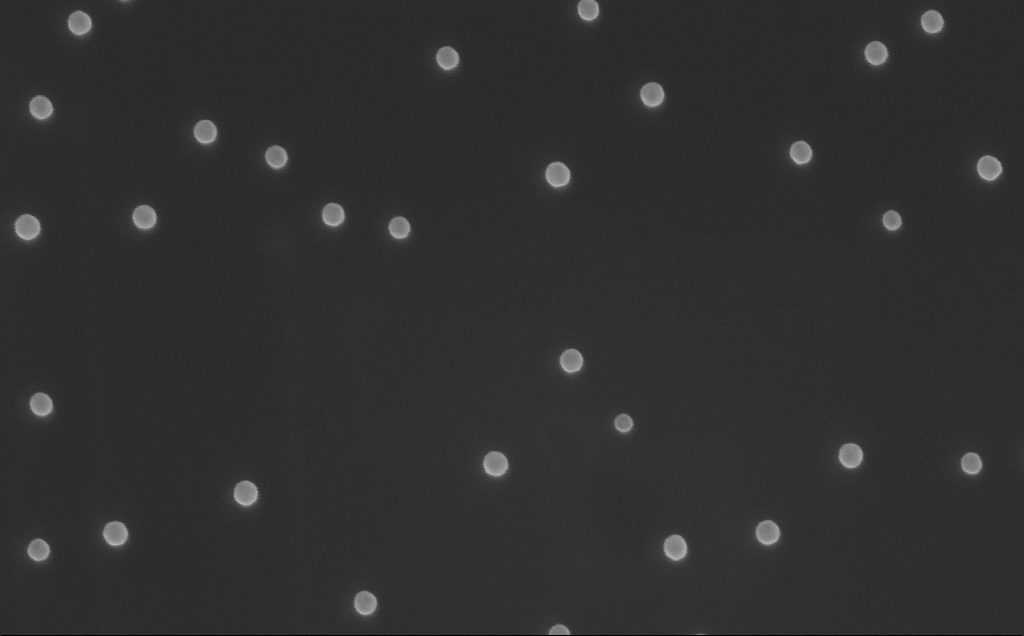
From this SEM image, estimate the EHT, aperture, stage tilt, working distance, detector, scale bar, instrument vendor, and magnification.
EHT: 10 kV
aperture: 30 µm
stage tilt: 0°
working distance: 8 mm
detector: InLens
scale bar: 100 nm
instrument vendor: Zeiss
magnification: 150 K X